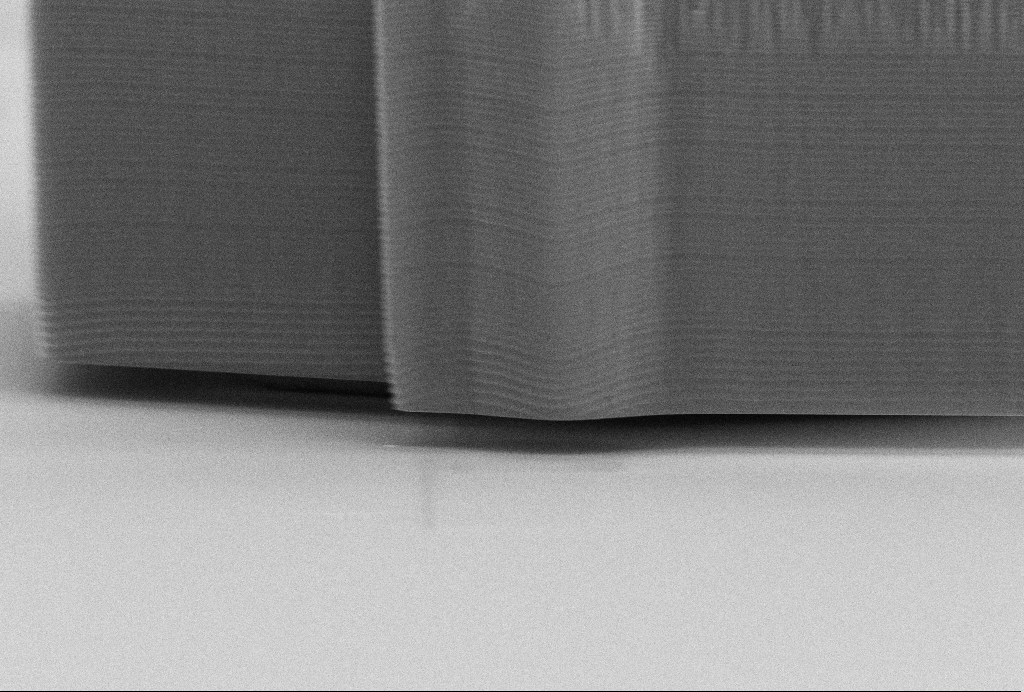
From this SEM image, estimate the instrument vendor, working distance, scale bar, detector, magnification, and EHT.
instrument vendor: Zeiss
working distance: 15.8 mm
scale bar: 2000 nm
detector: InLens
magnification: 13.22 K X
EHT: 10 kV